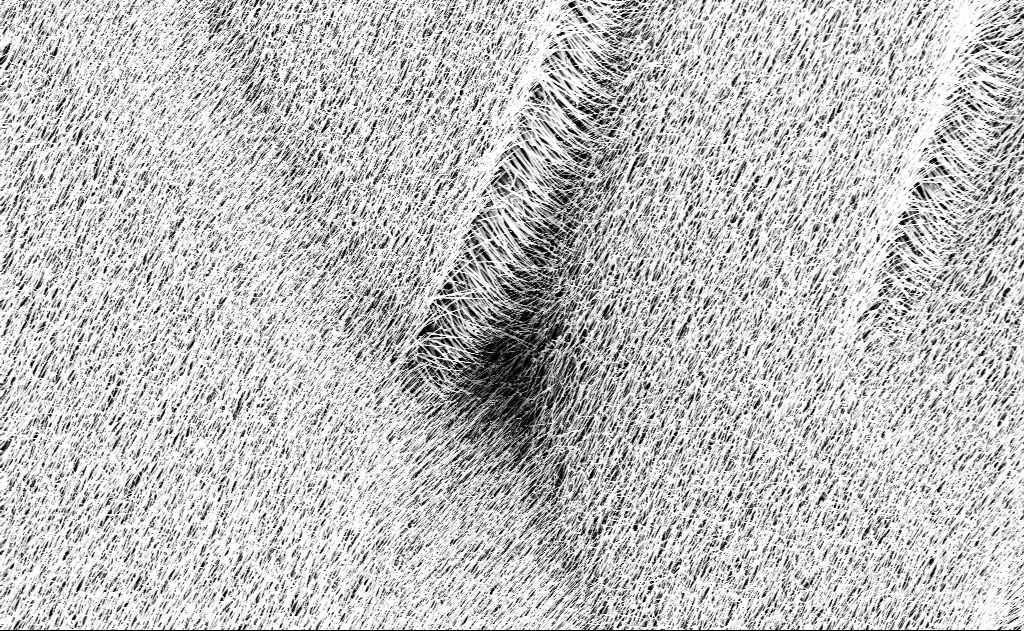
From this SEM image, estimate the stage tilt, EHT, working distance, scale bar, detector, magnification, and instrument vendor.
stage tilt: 0°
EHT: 10 kV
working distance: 13 mm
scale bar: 10000 nm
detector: InLens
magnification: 5 K X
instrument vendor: Zeiss